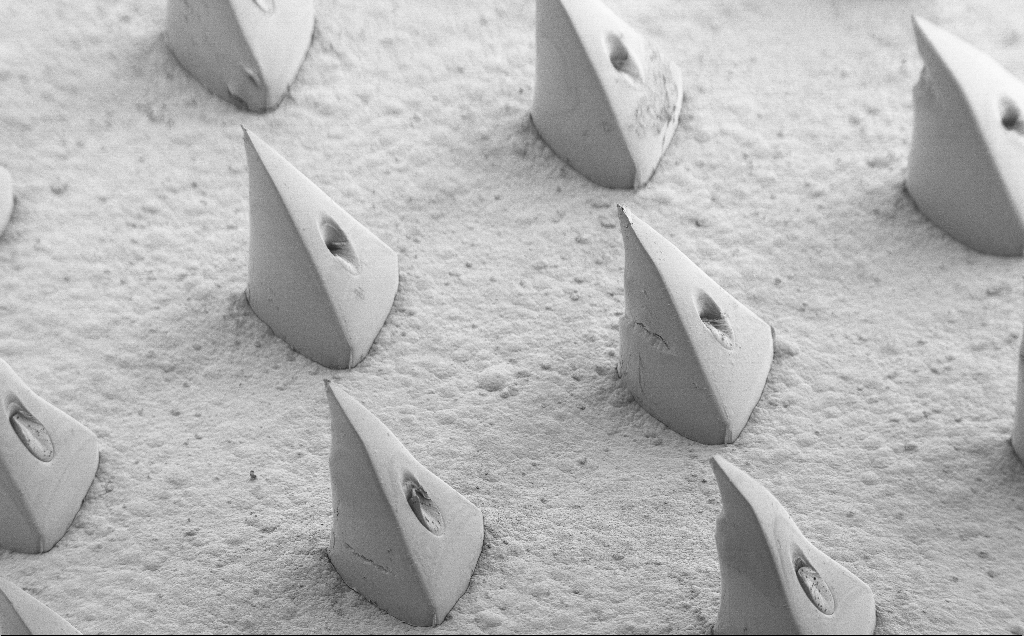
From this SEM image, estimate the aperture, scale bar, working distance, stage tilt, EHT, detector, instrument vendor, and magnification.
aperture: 30 µm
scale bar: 200000 nm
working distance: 8 mm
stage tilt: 40°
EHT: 5 kV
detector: SE2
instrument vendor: Zeiss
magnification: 0.098 K X